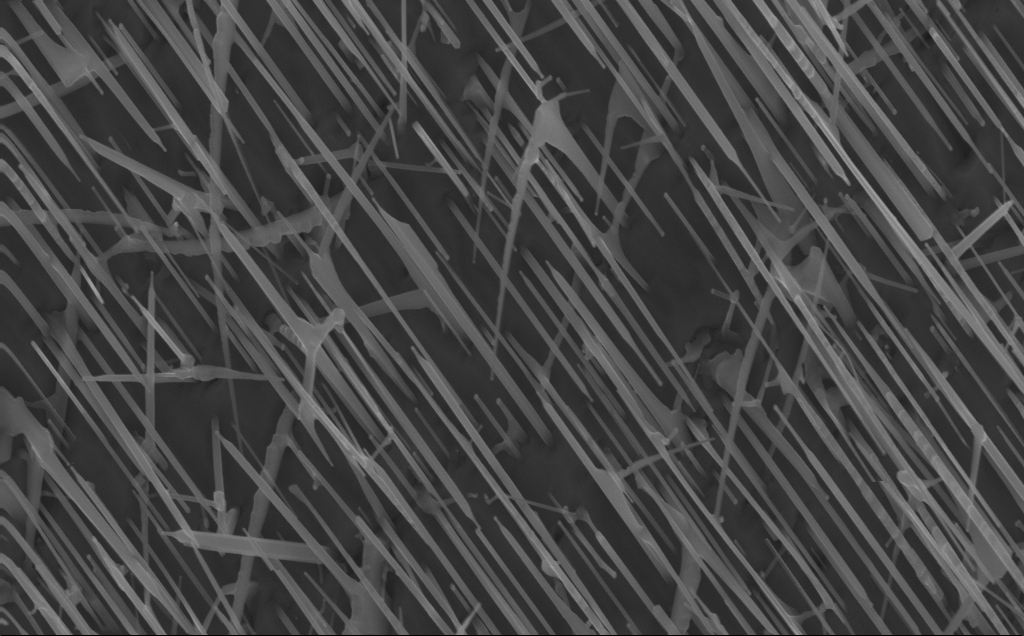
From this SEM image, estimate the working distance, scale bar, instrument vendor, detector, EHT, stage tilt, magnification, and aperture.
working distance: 4 mm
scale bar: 1000 nm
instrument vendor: Zeiss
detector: InLens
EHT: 10 kV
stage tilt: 0°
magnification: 40 K X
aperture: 30 µm